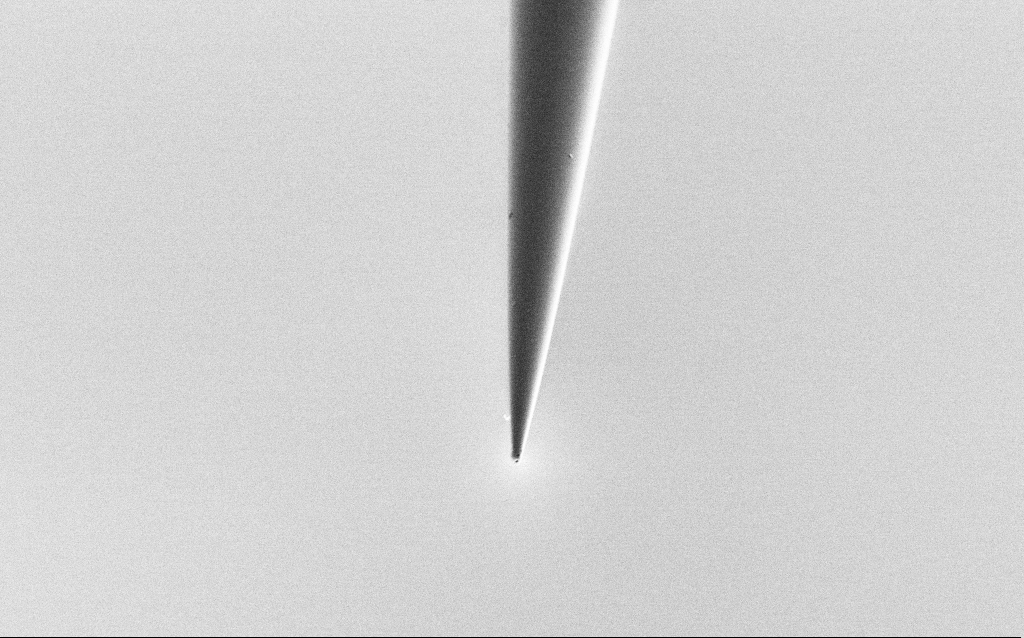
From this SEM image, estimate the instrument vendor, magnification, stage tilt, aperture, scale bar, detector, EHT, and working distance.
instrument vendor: Zeiss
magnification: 10 K X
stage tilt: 45°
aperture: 30 µm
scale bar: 2000 nm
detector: SE2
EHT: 2 kV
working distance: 6 mm